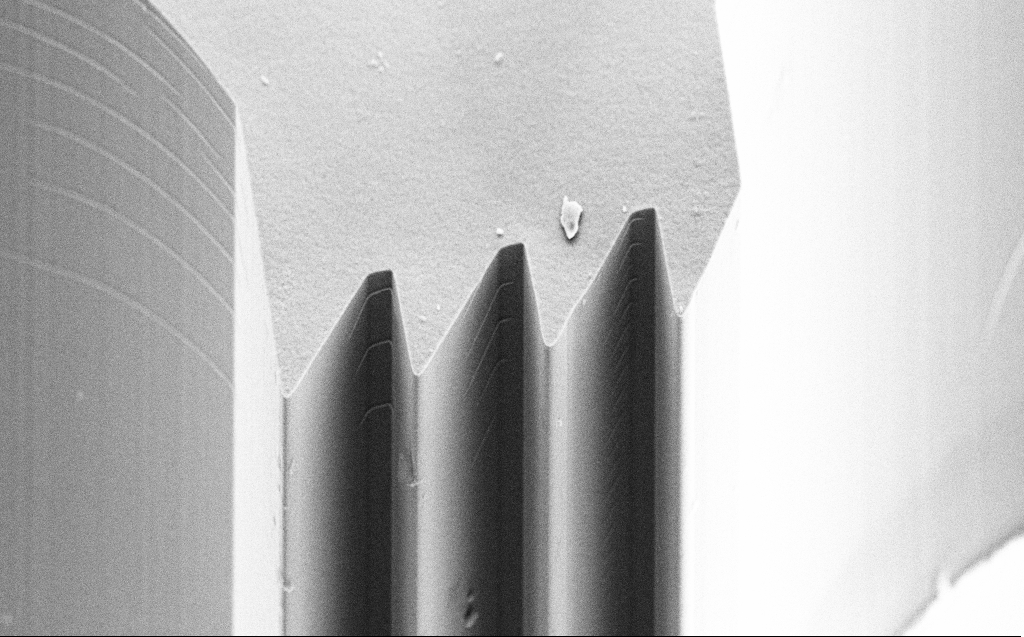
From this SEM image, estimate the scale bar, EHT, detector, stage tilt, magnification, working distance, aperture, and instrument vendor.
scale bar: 10000 nm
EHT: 10 kV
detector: SE2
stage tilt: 45°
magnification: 4.33 K X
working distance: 9 mm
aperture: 30 µm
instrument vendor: Zeiss